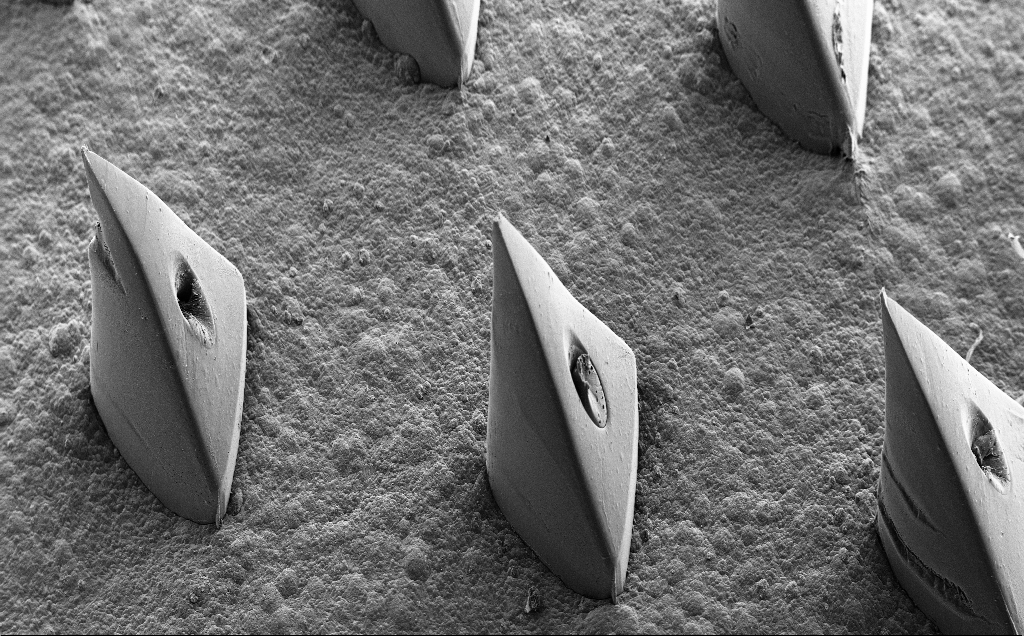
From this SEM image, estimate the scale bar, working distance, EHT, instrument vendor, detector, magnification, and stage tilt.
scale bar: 200000 nm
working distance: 11 mm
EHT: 10 kV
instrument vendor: Zeiss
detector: SE2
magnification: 0.096 K X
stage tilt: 35.3°